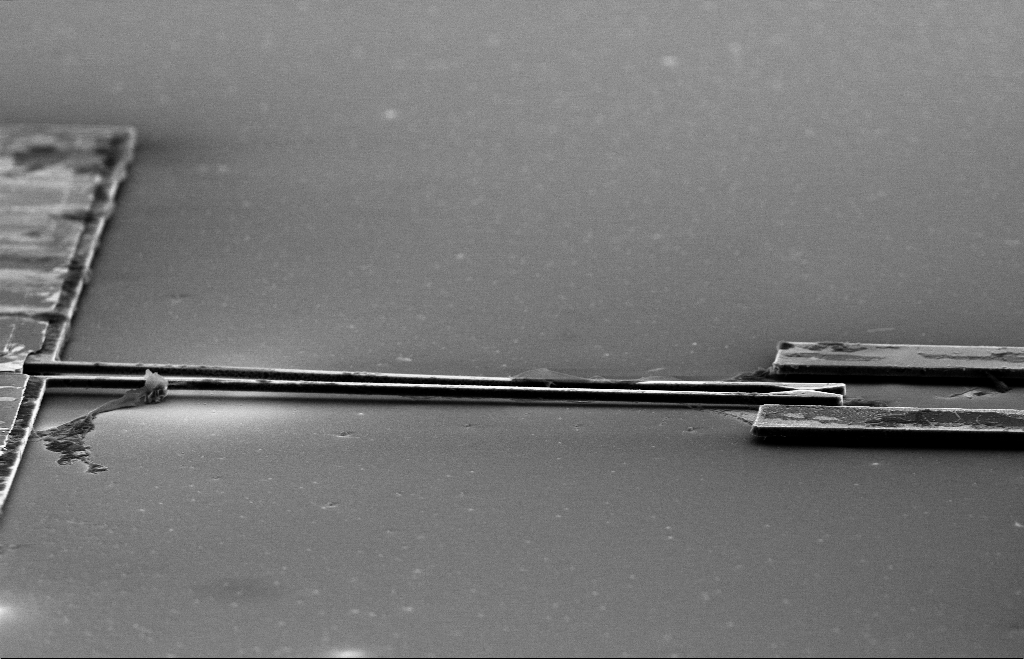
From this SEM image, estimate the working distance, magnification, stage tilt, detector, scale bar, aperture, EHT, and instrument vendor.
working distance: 11 mm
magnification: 1.47 K X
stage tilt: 70°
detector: SE2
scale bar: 20000 nm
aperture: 30 µm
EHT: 10 kV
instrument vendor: Zeiss